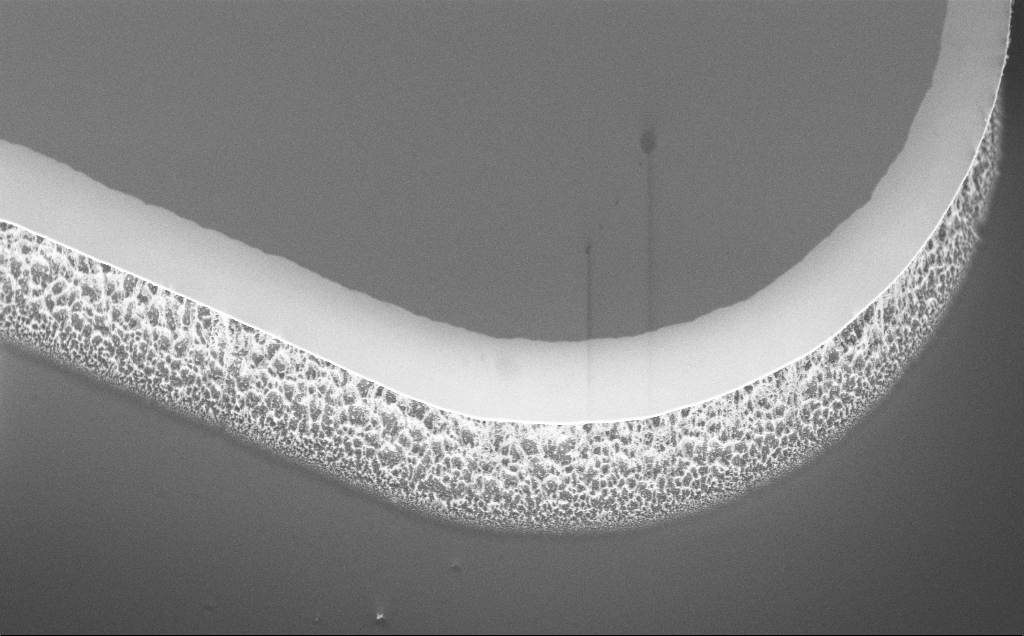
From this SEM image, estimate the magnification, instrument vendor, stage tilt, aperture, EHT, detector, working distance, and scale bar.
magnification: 4.17 K X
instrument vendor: Zeiss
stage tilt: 45°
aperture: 30 µm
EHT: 5 kV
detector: InLens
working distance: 7 mm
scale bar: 10000 nm